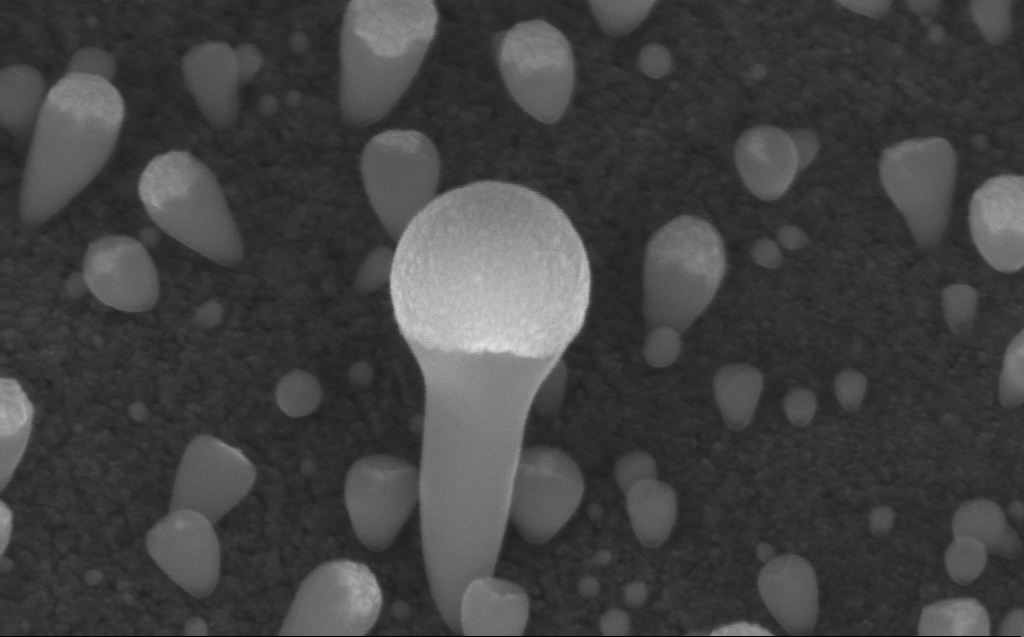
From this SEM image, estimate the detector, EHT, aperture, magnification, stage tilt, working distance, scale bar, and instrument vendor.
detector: InLens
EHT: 10 kV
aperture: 30 µm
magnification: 200 K X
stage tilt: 45°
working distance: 7 mm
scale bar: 100 nm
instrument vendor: Zeiss